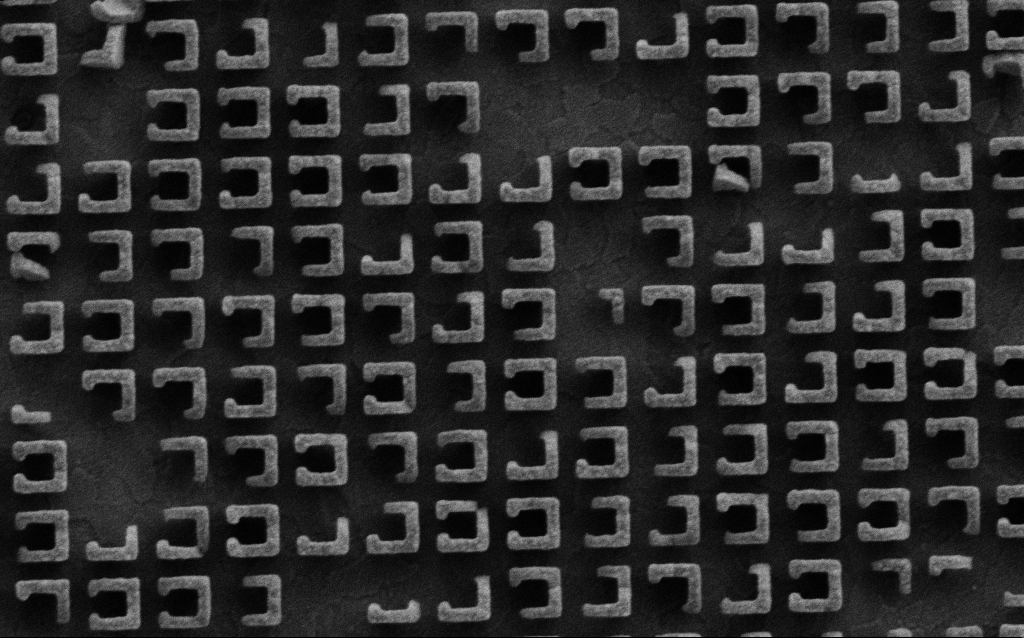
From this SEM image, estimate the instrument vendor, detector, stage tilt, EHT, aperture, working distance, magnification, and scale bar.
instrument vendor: Zeiss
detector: SE2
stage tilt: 0°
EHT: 3 kV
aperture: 30 µm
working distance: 6.5 mm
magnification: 55.87 K X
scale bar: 1000 nm